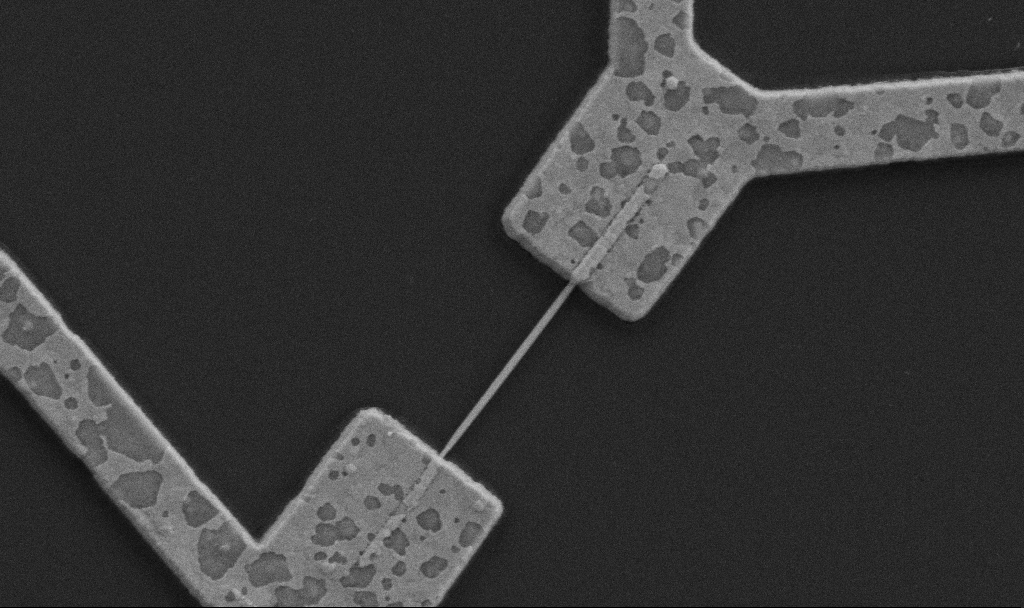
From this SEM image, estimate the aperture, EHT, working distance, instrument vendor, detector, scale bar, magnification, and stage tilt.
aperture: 30 µm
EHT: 5 kV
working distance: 10.7 mm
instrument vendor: Zeiss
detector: SE2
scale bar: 1000 nm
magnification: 30 K X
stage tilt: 0°